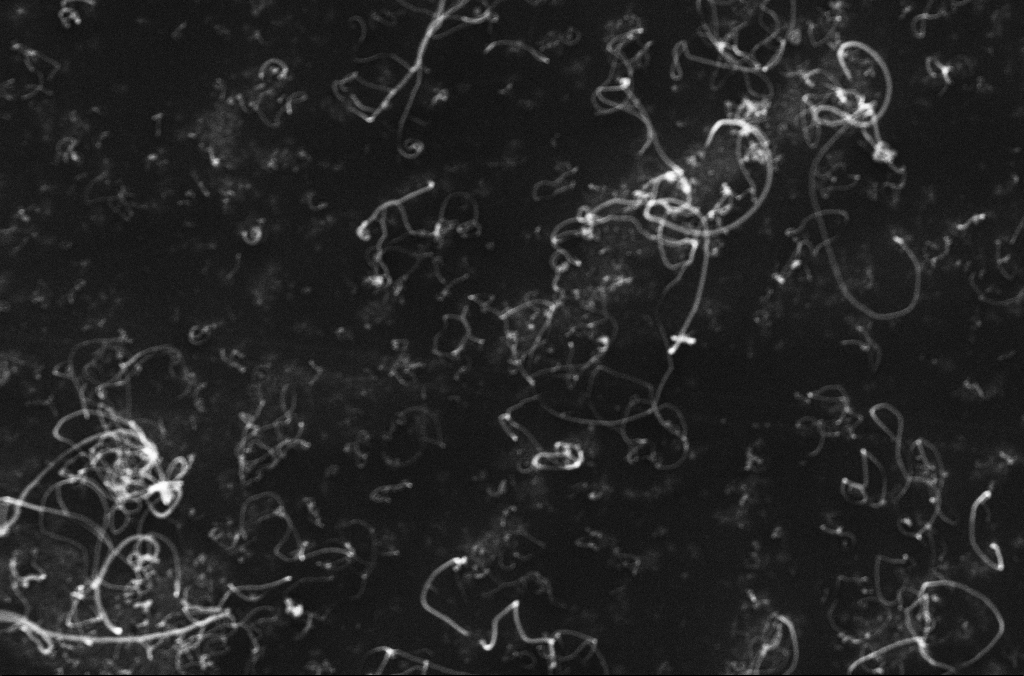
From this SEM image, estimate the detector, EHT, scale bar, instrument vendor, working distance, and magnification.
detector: InLens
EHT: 10 kV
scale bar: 2000 nm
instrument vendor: Zeiss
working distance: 3.3 mm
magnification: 30 K X